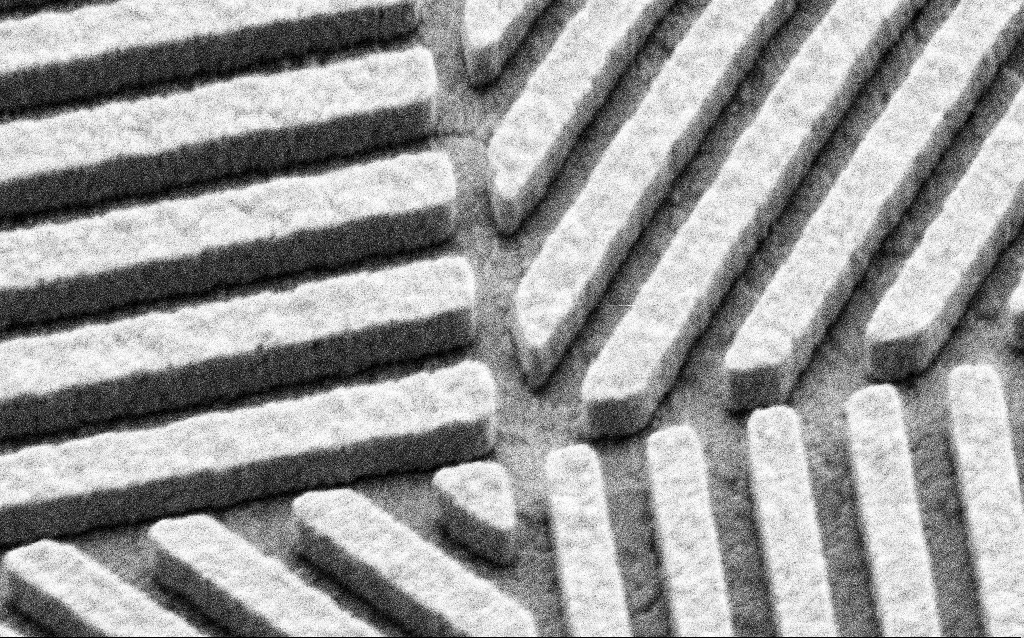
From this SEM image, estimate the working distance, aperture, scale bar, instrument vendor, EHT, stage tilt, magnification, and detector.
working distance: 6.2 mm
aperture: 30 µm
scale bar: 200 nm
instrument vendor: Zeiss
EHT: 3 kV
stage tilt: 45°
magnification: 94.1 K X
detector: SE2